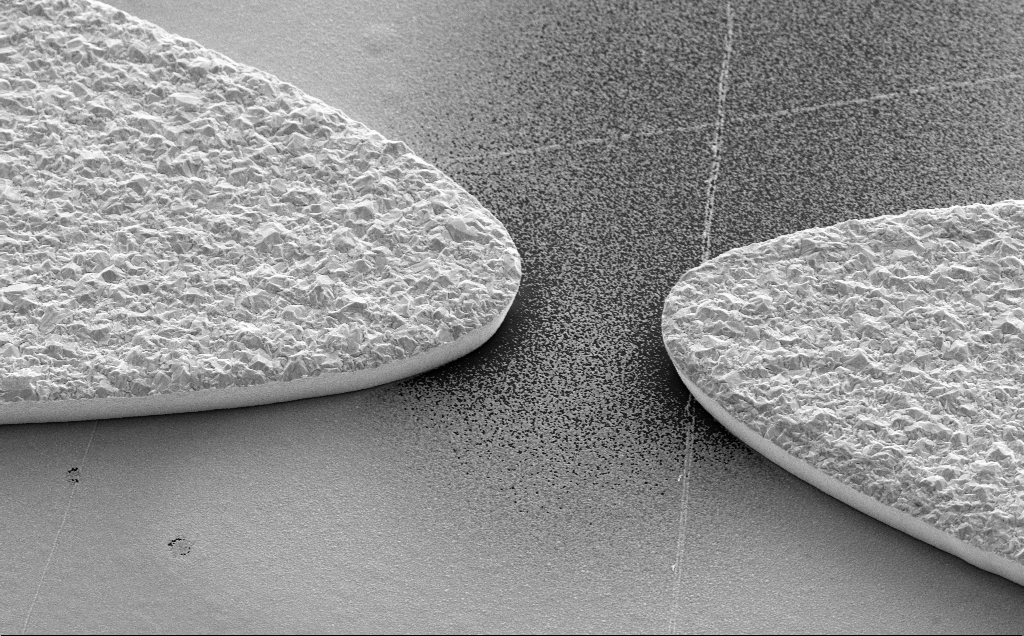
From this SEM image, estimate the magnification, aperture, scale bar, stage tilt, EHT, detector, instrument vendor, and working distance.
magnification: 11.95 K X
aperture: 30 µm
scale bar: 2000 nm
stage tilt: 35°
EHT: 5 kV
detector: SE2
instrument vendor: Zeiss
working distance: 13 mm